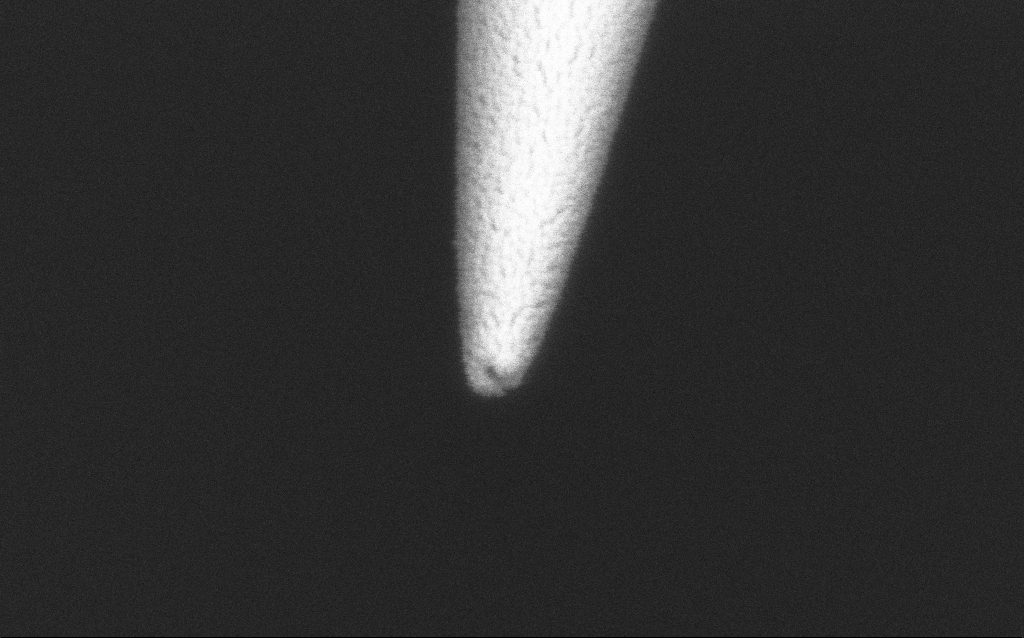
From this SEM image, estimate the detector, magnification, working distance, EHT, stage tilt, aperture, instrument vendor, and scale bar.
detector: InLens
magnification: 200 K X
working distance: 7.6 mm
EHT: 2 kV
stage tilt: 45°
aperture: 30 µm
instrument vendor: Zeiss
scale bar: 200 nm